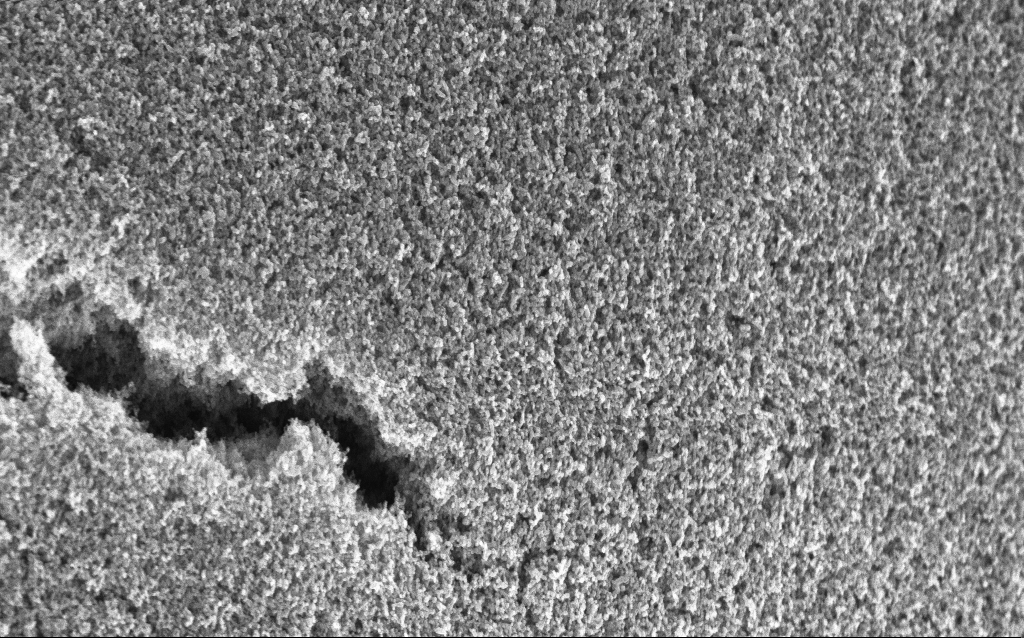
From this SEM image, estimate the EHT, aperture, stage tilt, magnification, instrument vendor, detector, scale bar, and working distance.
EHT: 5 kV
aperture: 30 µm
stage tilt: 0°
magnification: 65.04 K X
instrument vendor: Zeiss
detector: InLens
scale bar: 1000 nm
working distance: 2.6 mm